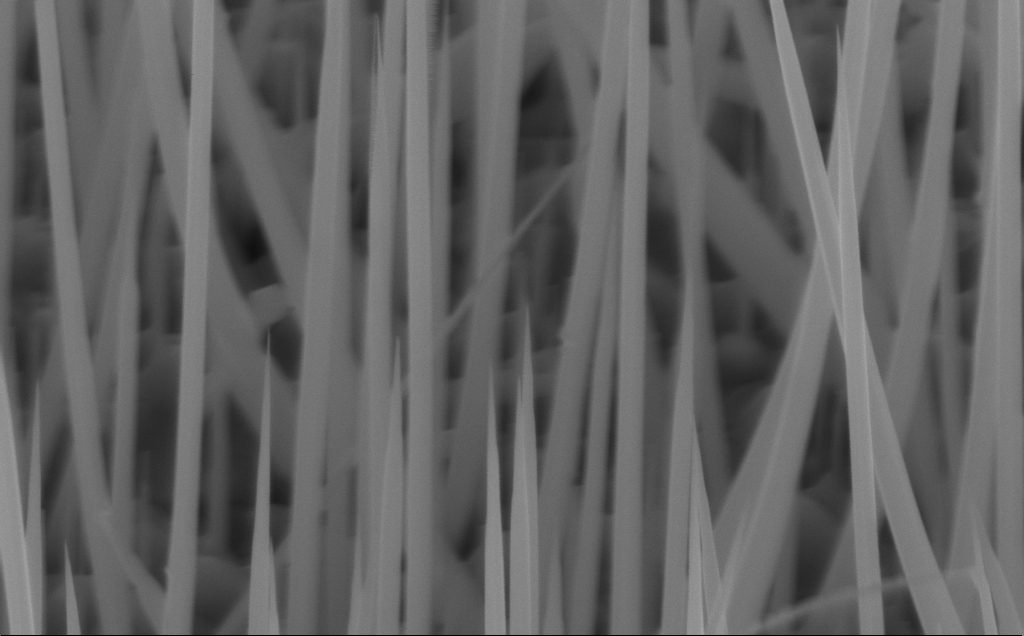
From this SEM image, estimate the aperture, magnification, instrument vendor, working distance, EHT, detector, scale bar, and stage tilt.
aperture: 30 µm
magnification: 80 K X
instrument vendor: Zeiss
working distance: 7 mm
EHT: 10 kV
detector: InLens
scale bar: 200 nm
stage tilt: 45°